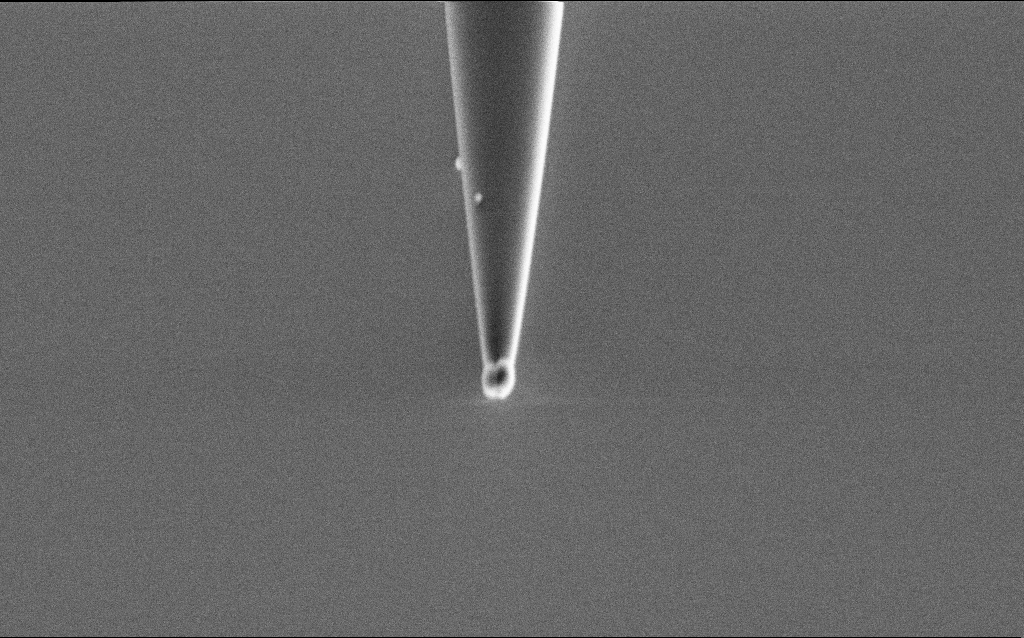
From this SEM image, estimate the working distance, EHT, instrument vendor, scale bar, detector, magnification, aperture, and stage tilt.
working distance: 6.5 mm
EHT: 2 kV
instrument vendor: Zeiss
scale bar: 1000 nm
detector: SE2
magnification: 50 K X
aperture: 30 µm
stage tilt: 44.9°